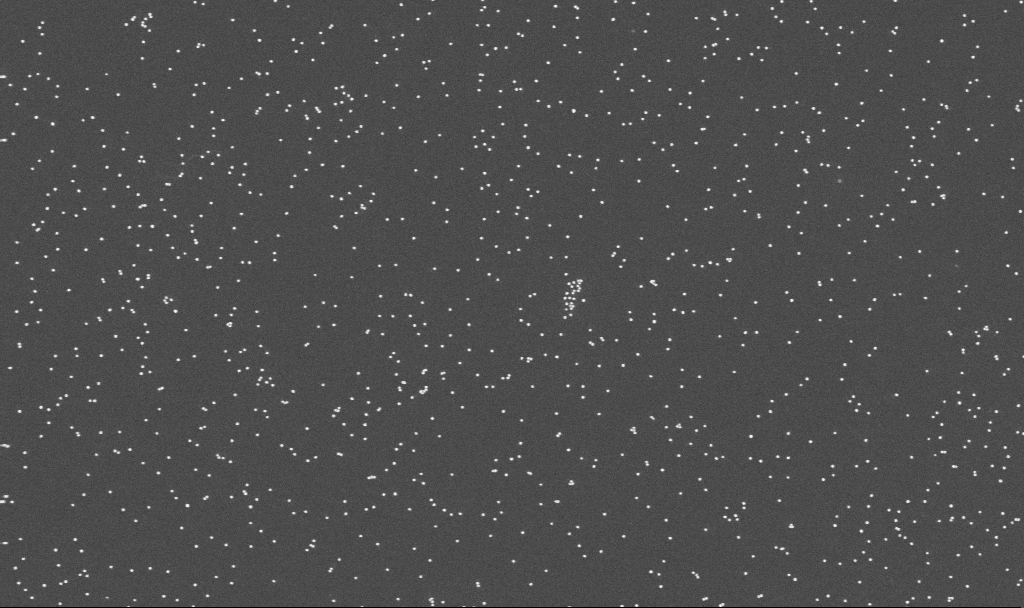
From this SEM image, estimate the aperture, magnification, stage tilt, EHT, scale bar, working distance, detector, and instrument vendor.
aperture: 30 µm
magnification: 100 K X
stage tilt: -0°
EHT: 10 kV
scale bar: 200 nm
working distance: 3.3 mm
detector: InLens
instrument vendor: Zeiss